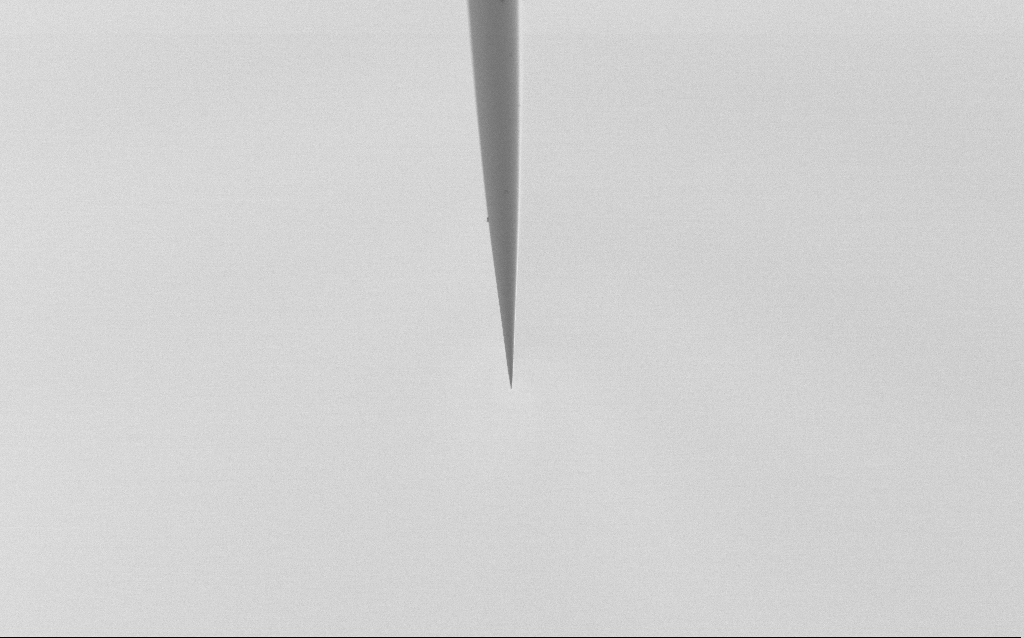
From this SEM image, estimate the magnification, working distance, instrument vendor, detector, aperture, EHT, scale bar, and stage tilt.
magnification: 1 K X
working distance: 5 mm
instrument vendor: Zeiss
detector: SE2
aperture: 30 µm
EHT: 2.5 kV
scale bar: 20000 nm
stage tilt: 45°